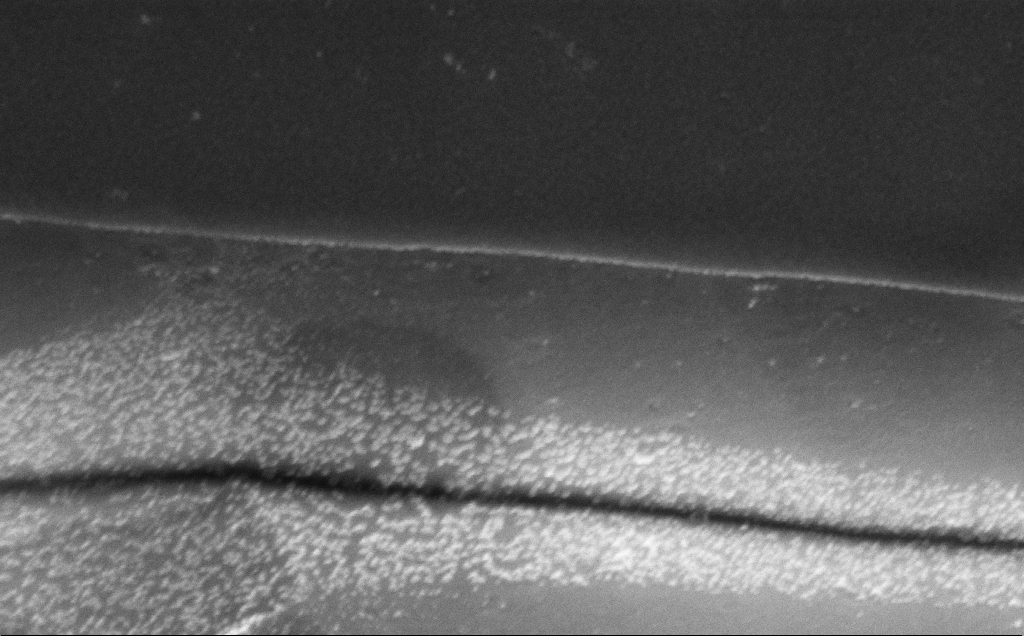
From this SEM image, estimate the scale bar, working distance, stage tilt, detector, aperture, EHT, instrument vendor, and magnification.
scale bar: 1000 nm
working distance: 14 mm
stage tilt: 0°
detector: InLens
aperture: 30 µm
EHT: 5 kV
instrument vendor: Zeiss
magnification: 61.68 K X